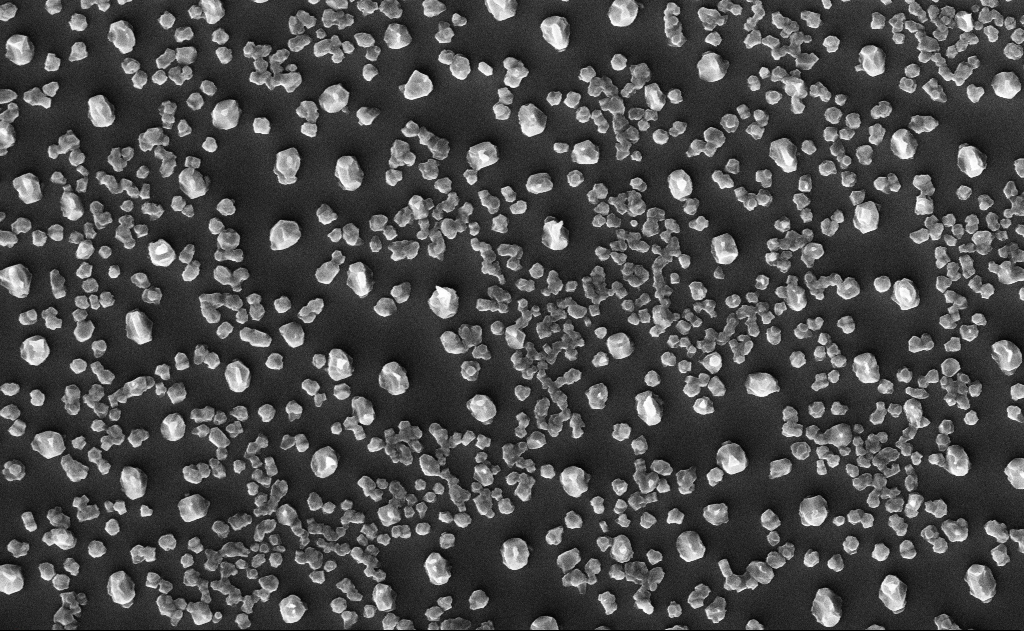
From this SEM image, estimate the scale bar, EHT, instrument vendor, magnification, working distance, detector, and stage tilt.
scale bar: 2000 nm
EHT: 10 kV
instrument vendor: Zeiss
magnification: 20 K X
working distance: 18 mm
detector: InLens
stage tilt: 0°